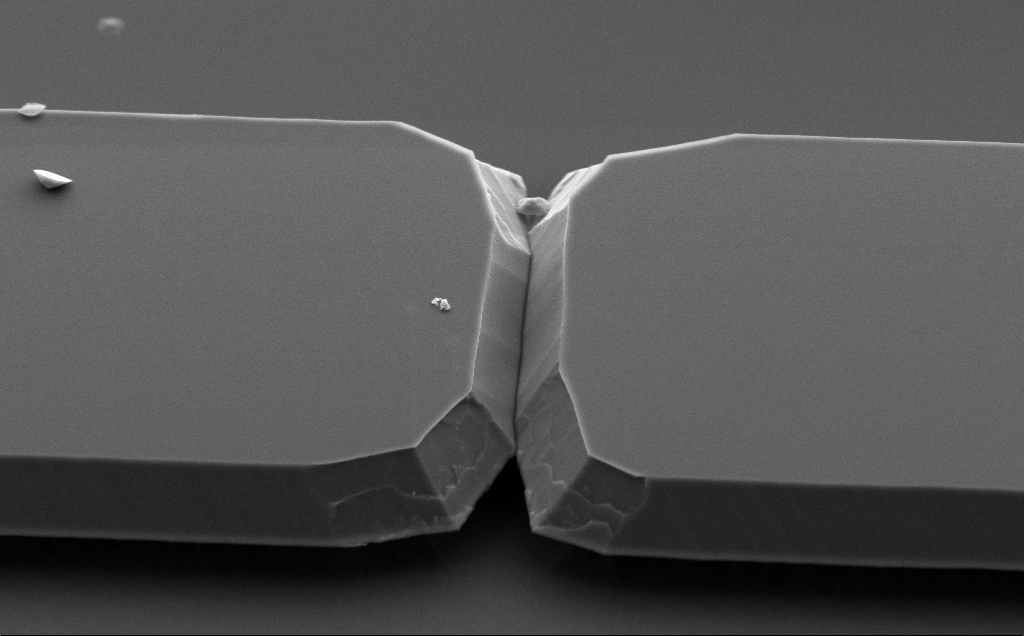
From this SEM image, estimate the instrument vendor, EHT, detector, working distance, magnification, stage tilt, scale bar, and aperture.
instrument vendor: Zeiss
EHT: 5 kV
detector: SE2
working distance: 10 mm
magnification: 10.51 K X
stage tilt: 50°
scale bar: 2000 nm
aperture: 30 µm